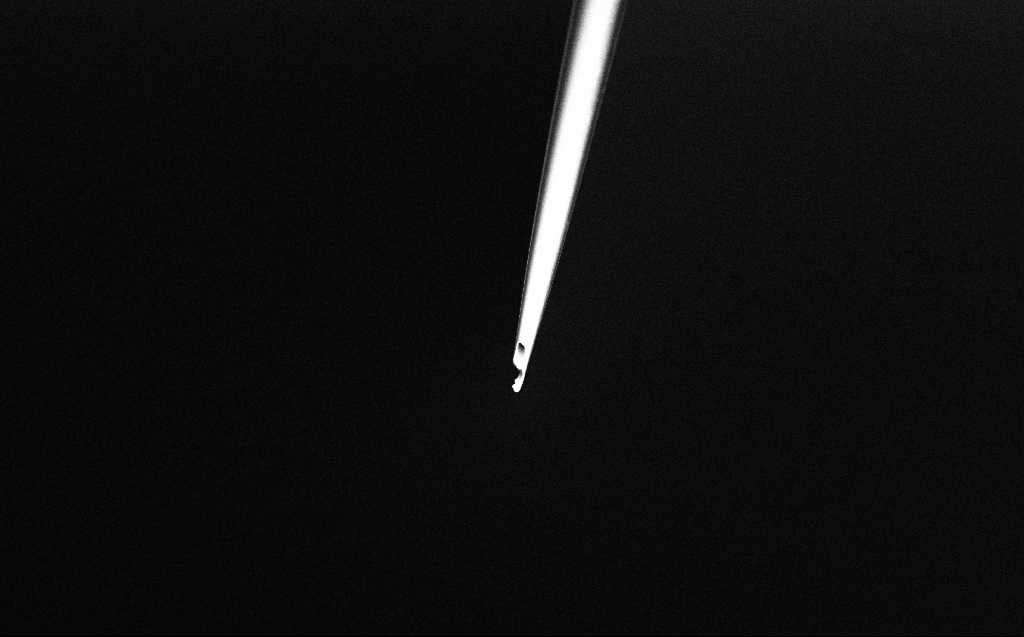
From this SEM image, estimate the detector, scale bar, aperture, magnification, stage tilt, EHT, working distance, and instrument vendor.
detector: InLens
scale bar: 20000 nm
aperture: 30 µm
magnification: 1 K X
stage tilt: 45°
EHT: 2 kV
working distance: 5 mm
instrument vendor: Zeiss